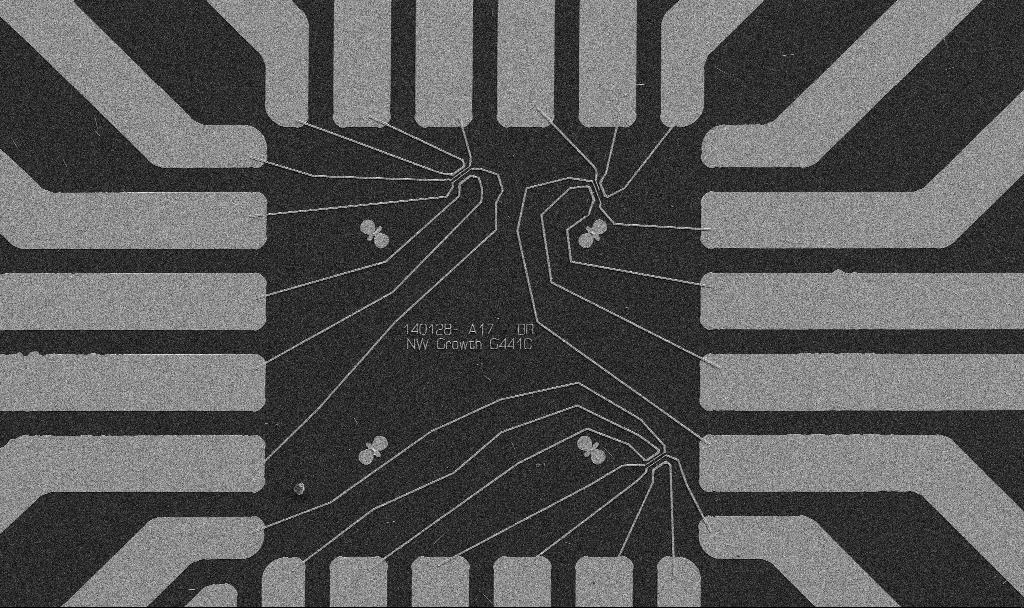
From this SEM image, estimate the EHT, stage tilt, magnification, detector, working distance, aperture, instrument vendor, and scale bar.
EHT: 5 kV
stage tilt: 0°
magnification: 1 K X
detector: SE2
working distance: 10.7 mm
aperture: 30 µm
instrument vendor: Zeiss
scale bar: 20000 nm